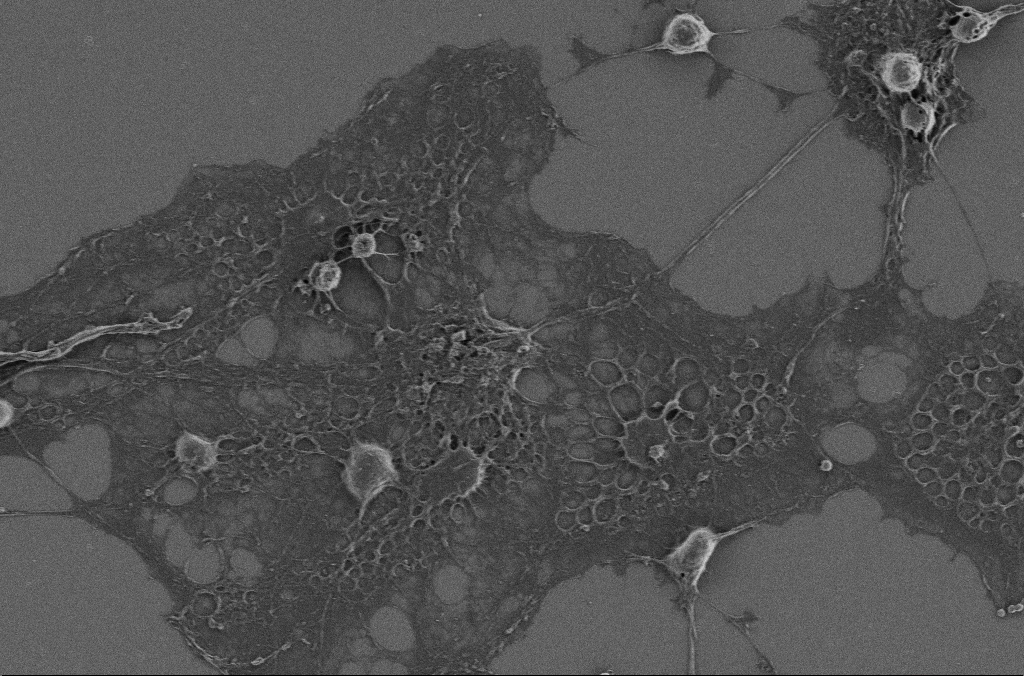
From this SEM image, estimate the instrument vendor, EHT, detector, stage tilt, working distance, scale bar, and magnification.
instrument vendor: Zeiss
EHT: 2 kV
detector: SE2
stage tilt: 0°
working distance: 3.8 mm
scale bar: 20000 nm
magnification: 2.5 K X